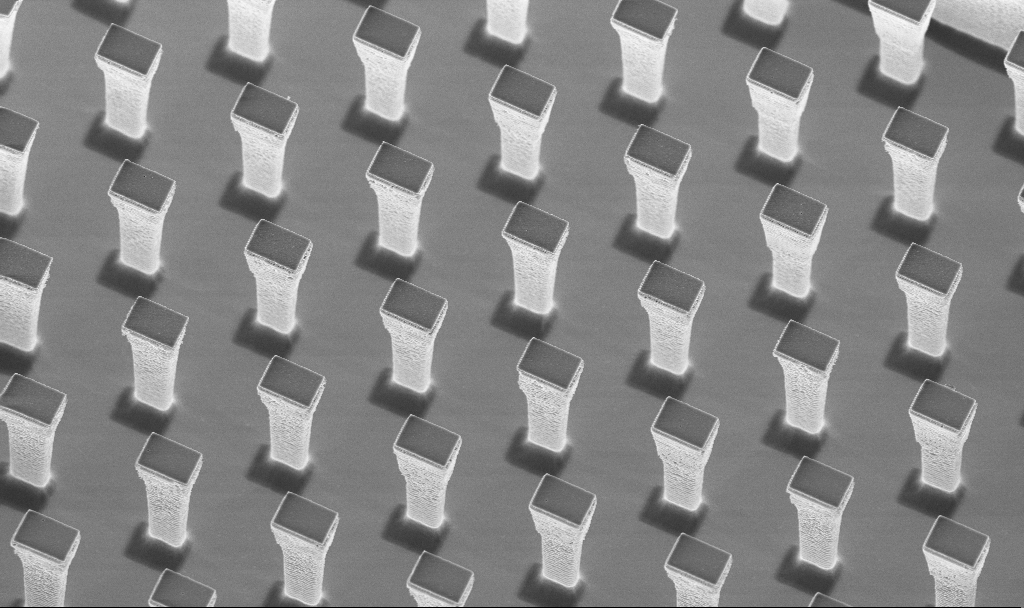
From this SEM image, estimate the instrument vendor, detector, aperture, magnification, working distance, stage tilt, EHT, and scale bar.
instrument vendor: Zeiss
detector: InLens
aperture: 30 µm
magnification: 4.6 K X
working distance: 4.2 mm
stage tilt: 20°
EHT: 5 kV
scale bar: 10000 nm